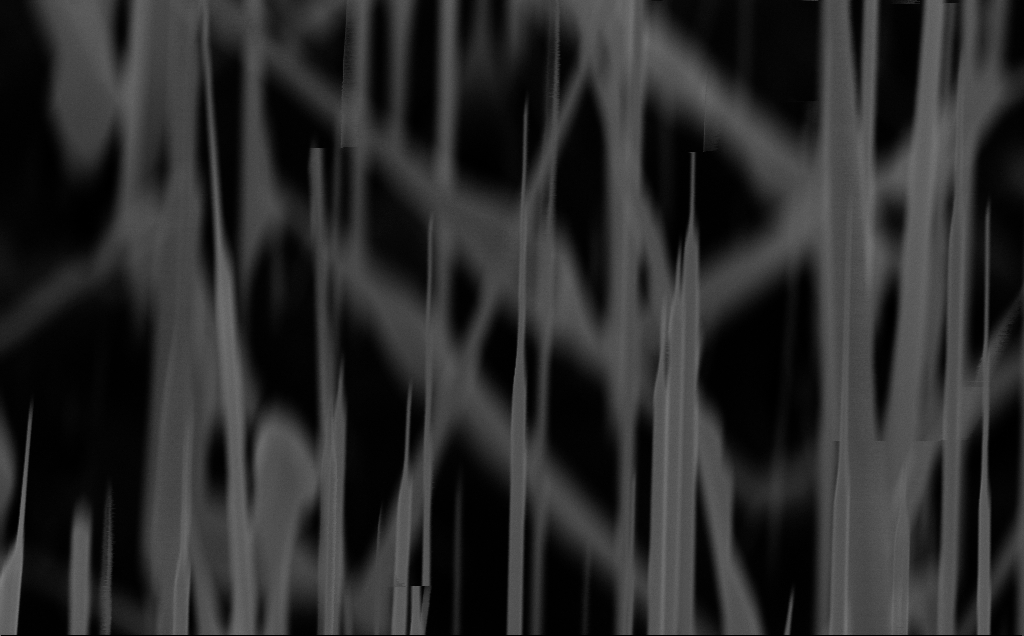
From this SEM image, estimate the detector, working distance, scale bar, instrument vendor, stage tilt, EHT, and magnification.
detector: InLens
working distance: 6 mm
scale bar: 1000 nm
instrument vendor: Zeiss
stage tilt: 45°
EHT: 10 kV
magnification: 66.65 K X